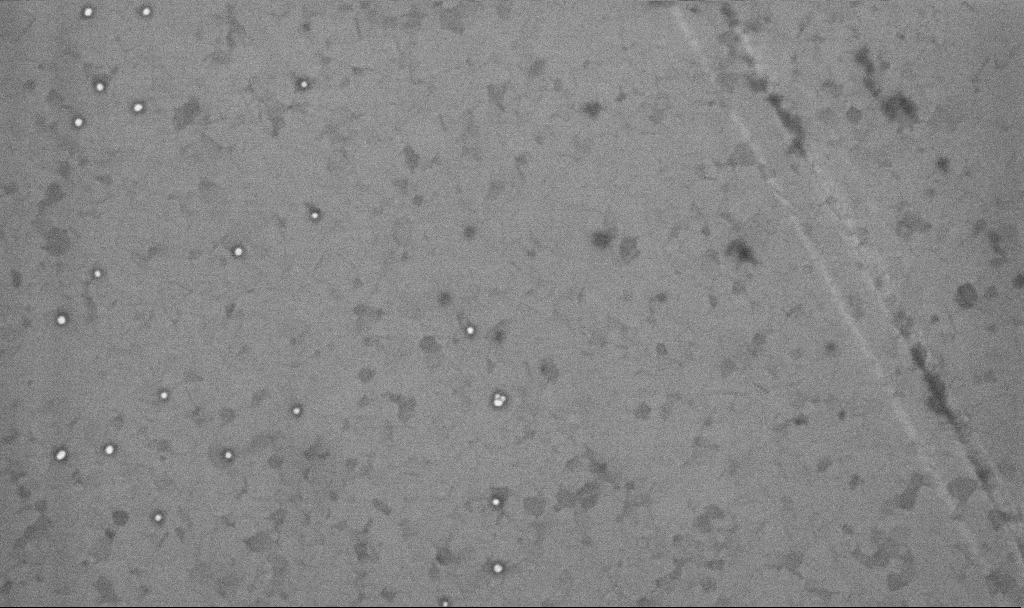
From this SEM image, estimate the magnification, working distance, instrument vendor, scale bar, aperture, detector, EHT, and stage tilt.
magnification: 100.38 K X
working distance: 3.3 mm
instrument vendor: Zeiss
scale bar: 200 nm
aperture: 30 µm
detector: InLens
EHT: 10 kV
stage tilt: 0°